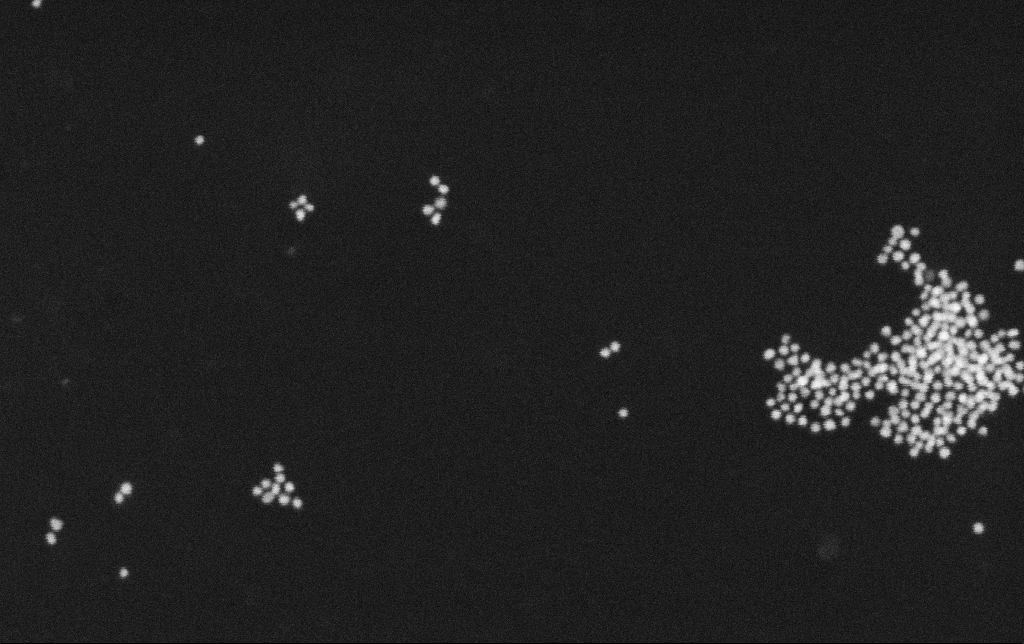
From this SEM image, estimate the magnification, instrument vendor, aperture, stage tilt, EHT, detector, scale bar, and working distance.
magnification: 310.47 K X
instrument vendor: Zeiss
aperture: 30 µm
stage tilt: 0°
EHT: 30 kV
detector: SE2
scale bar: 100 nm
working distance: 10.8 mm